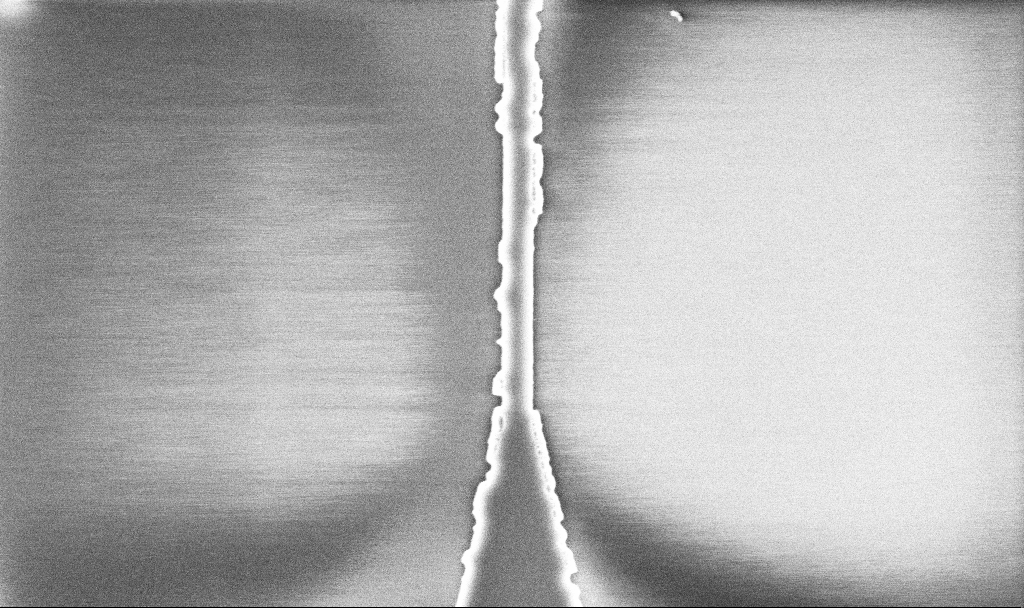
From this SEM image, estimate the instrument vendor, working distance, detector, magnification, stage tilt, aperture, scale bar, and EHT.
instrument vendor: Zeiss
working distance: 10.1 mm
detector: InLens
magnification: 20.81 K X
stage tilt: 0°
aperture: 30 µm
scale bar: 2000 nm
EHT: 5 kV